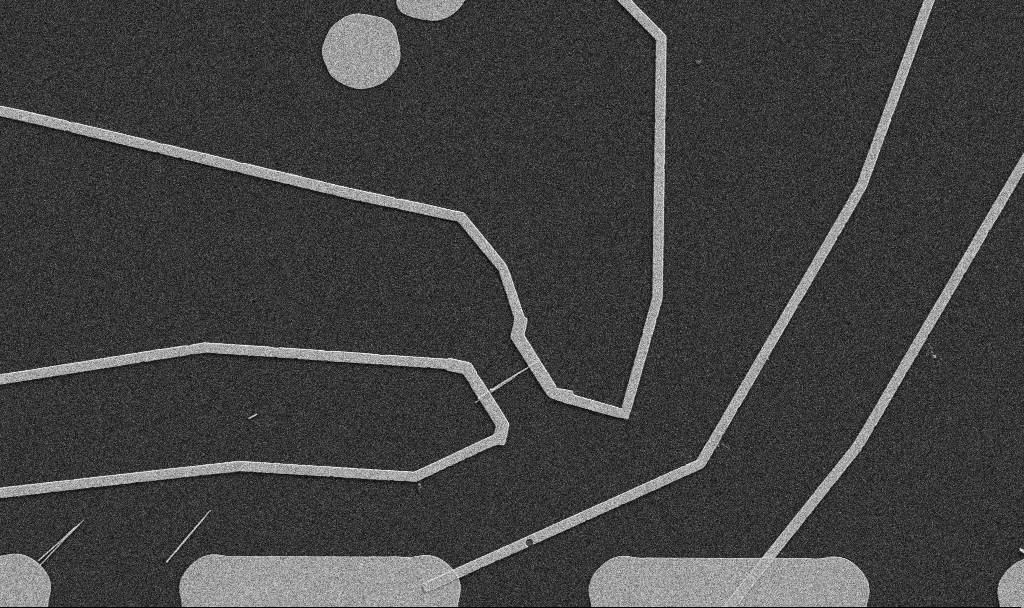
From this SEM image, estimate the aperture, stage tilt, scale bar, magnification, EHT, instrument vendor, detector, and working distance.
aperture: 30 µm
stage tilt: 0°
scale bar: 10000 nm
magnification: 5 K X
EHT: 5 kV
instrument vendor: Zeiss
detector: SE2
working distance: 10.7 mm